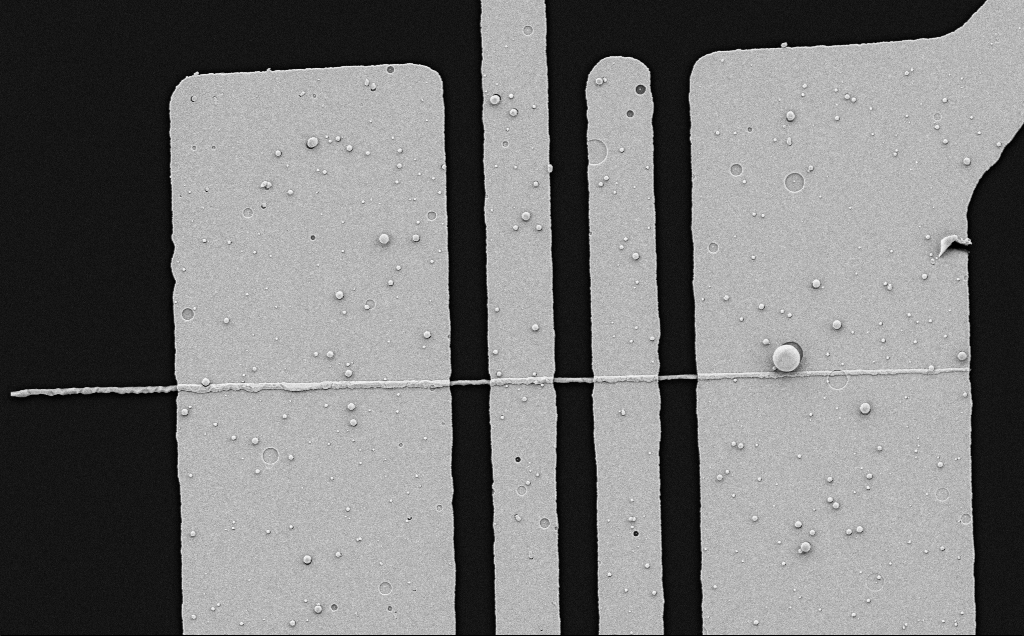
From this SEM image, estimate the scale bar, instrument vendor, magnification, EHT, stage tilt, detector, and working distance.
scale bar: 2000 nm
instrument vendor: Zeiss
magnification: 9.54 K X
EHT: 5 kV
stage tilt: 0°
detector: SE2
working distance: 10 mm